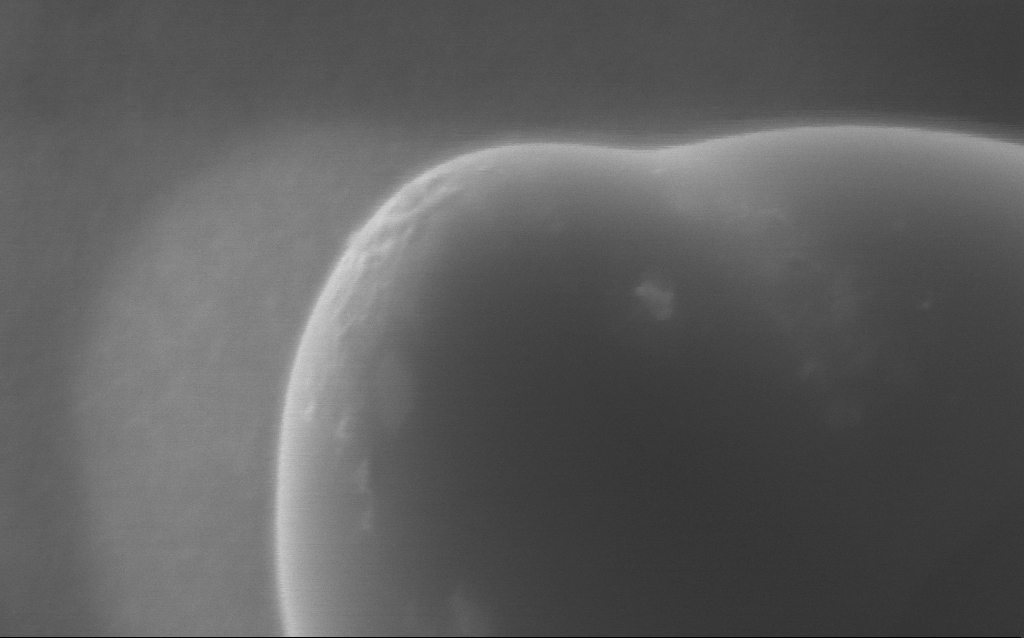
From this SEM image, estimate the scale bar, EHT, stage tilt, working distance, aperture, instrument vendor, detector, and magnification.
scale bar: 200 nm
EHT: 5 kV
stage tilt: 0°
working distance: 3 mm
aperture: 30 µm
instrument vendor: Zeiss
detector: InLens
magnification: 269 K X